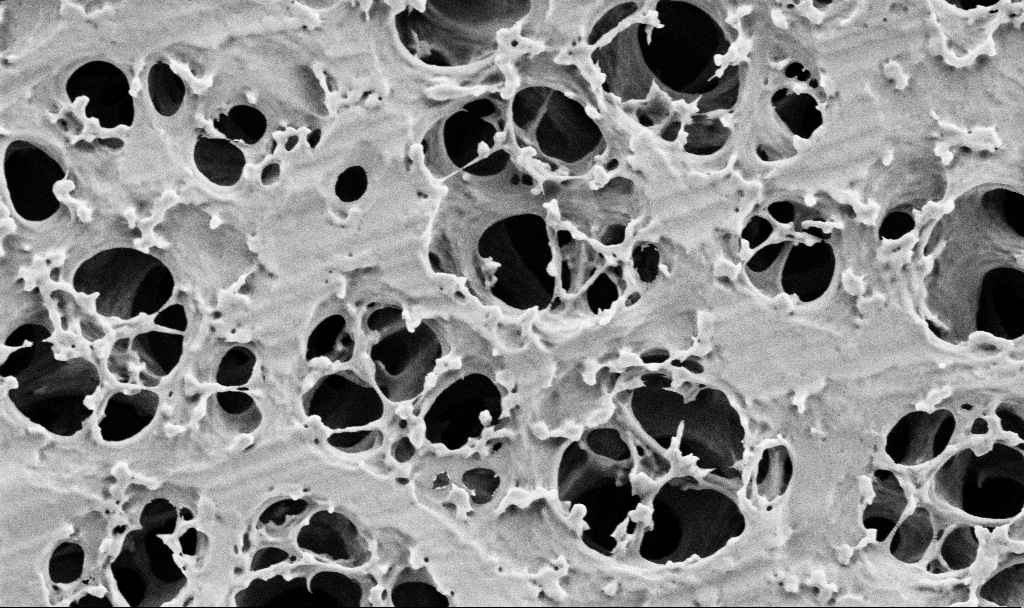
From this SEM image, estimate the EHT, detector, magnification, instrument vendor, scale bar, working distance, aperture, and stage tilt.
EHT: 2 kV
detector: SE2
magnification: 25 K X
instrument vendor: Zeiss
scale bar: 2000 nm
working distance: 3.5 mm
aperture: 30 µm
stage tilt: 0°